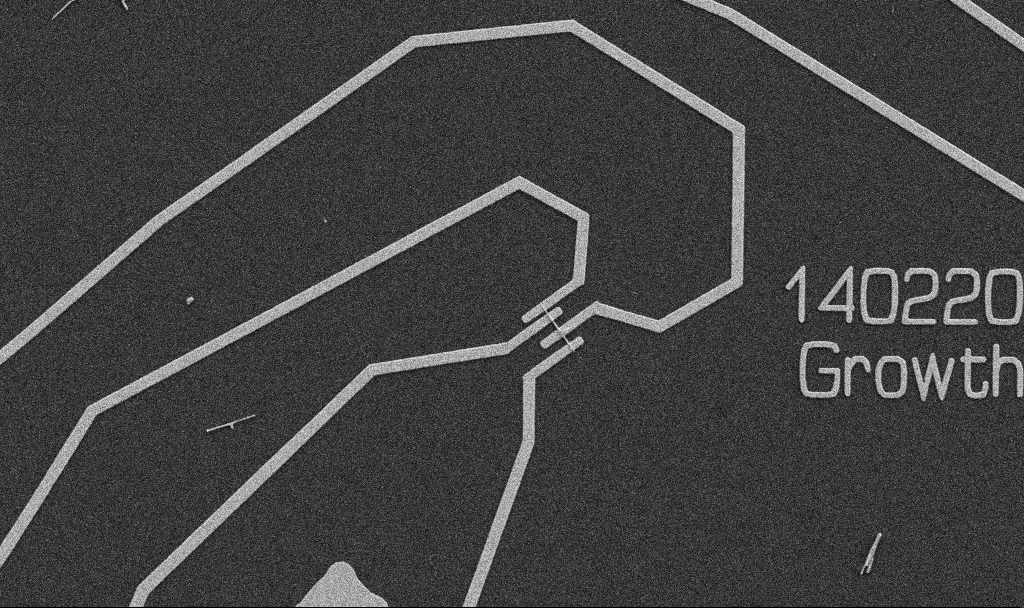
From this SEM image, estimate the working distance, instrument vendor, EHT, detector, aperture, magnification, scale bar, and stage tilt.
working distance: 10.7 mm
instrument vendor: Zeiss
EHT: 5 kV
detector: SE2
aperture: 30 µm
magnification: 5 K X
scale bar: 10000 nm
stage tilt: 0°